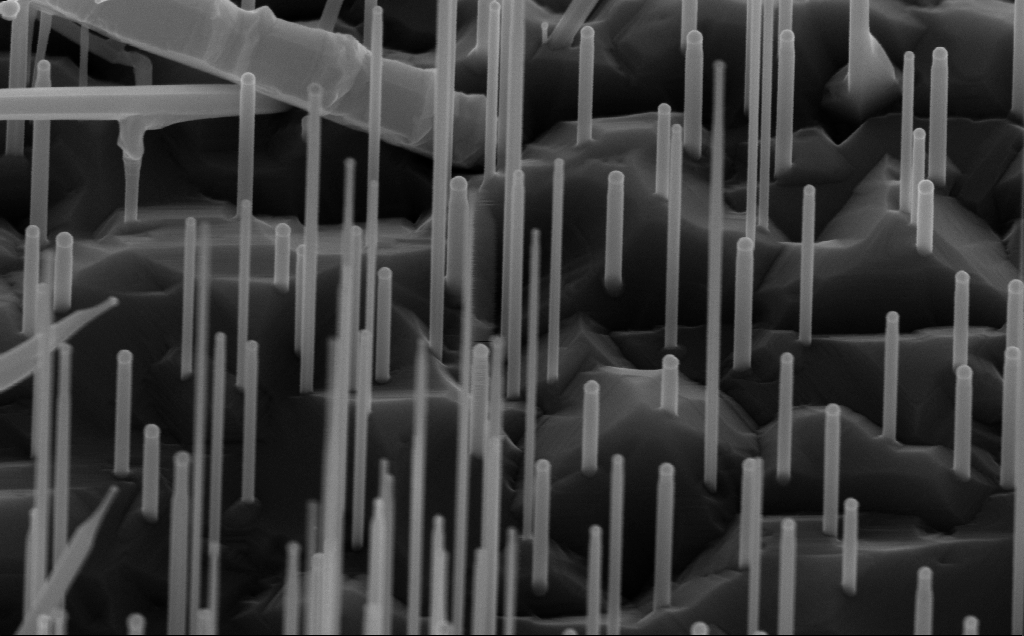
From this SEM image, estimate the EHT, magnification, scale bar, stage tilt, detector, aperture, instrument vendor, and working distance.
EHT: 10 kV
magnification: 87.35 K X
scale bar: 200 nm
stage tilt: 45°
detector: InLens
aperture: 30 µm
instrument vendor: Zeiss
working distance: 7 mm